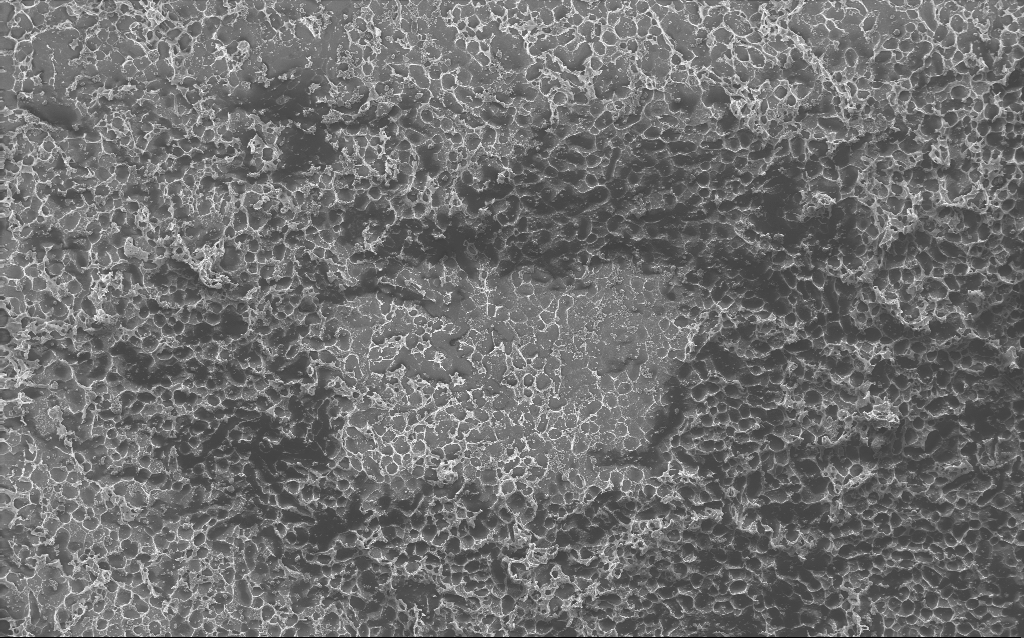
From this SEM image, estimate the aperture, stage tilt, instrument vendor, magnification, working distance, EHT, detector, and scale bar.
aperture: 30 µm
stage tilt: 0°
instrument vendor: Zeiss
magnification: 0.235 K X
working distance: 2.8 mm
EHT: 10 kV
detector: InLens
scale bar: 100000 nm